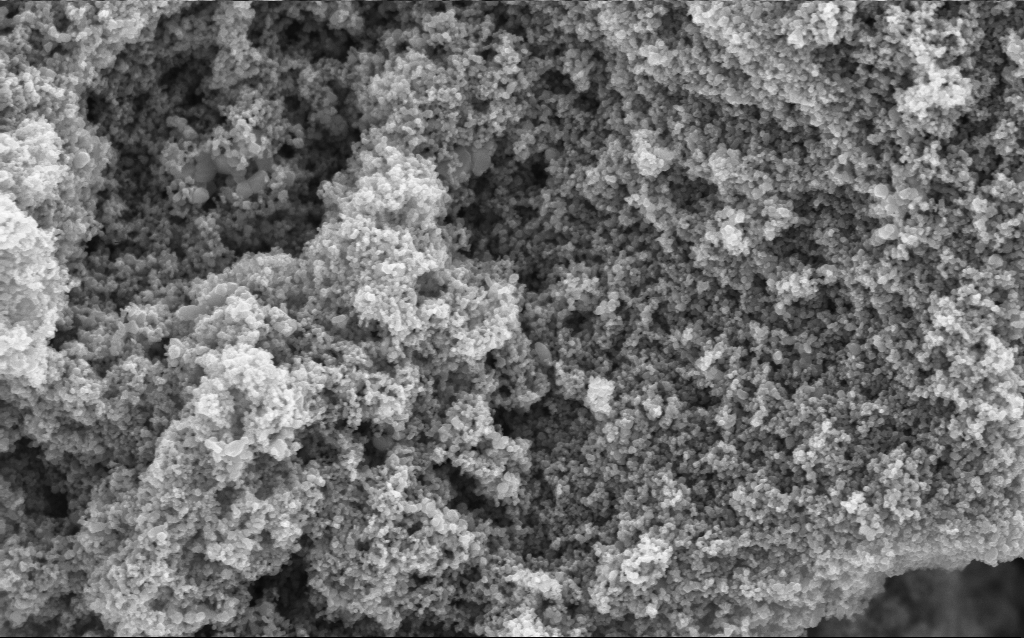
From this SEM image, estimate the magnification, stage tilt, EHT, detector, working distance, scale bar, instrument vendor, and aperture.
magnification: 68.65 K X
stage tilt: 0°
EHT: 5 kV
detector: InLens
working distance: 4.5 mm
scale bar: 1000 nm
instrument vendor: Zeiss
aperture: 30 µm